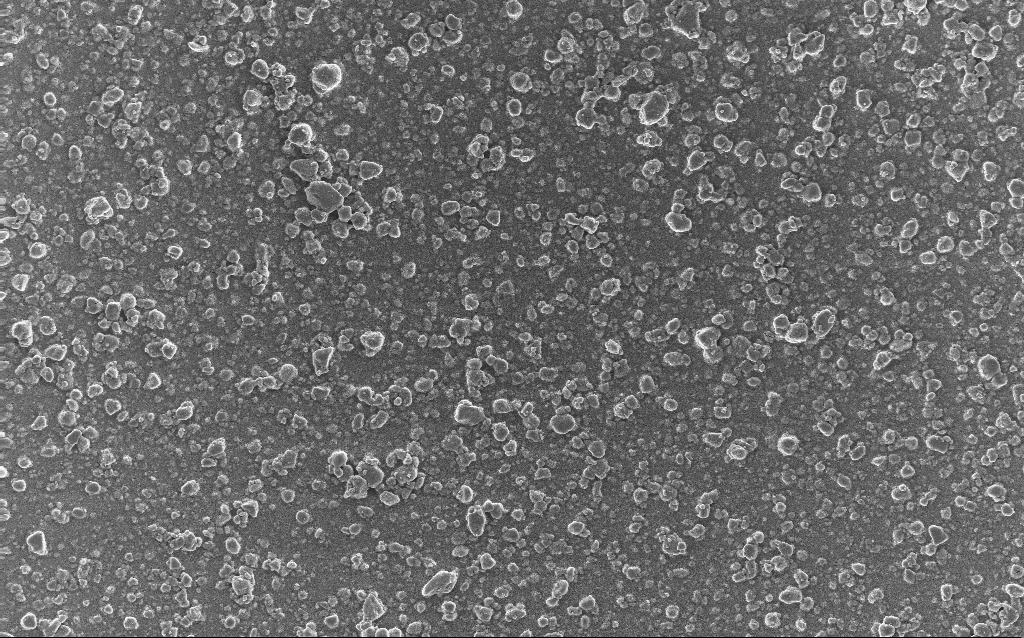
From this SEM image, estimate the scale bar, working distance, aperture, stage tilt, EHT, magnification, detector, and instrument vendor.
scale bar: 20000 nm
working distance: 2.7 mm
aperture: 30 µm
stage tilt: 0°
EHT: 10 kV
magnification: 0.77 K X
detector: InLens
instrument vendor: Zeiss